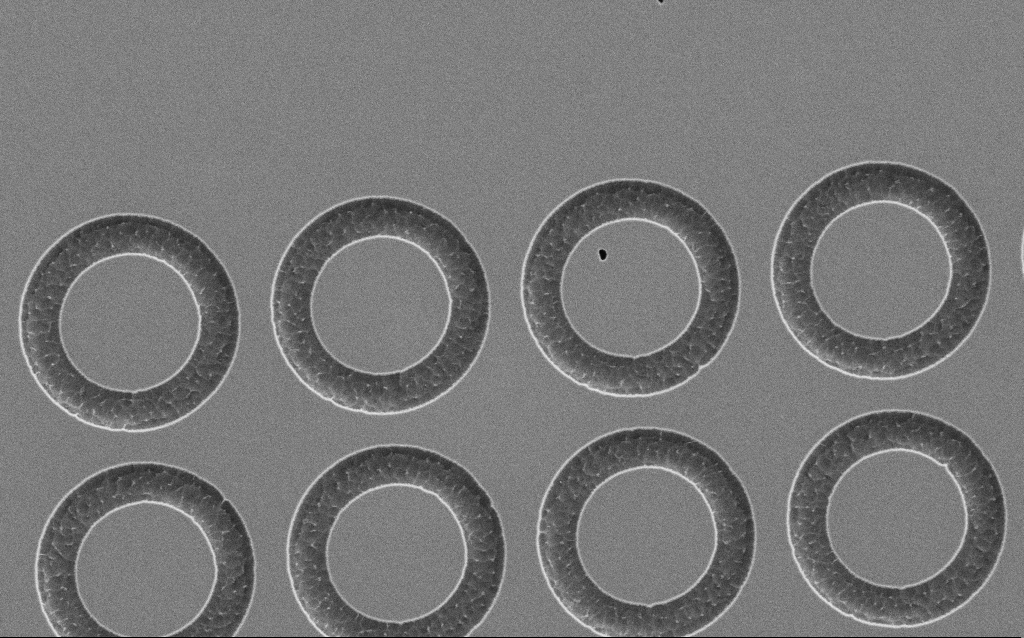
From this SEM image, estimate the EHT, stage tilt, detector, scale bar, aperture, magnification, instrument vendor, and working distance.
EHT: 2 kV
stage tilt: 0°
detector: SE2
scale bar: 1000 nm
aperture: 30 µm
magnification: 13.14 K X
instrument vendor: Zeiss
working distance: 5 mm